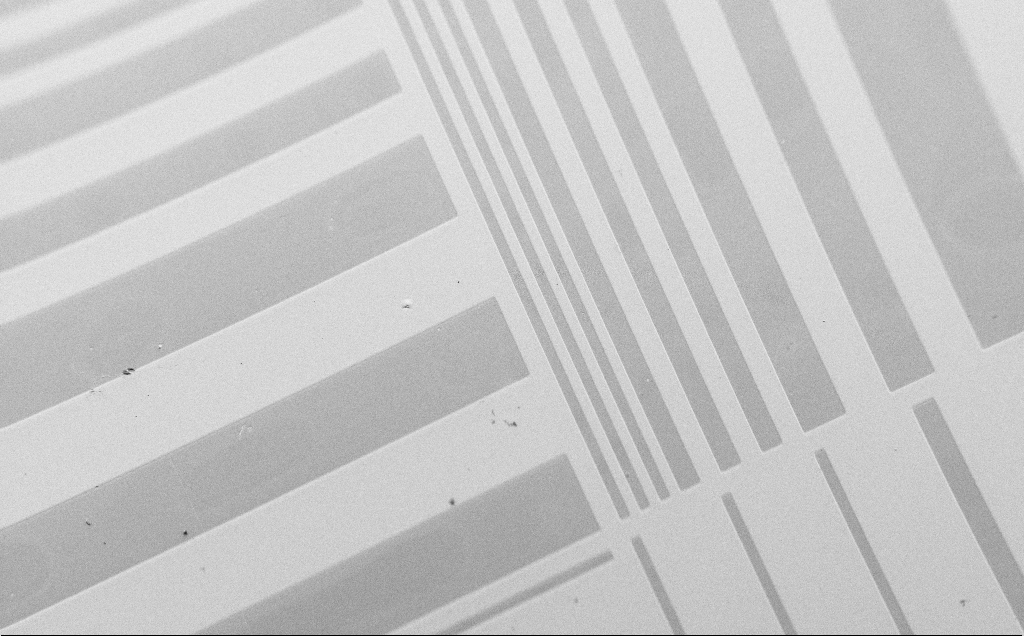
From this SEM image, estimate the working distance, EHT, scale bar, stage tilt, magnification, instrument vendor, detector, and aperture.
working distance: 4 mm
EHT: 1.5 kV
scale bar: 200000 nm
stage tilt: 45°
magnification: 0.164 K X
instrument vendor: Zeiss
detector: SE2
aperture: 30 µm